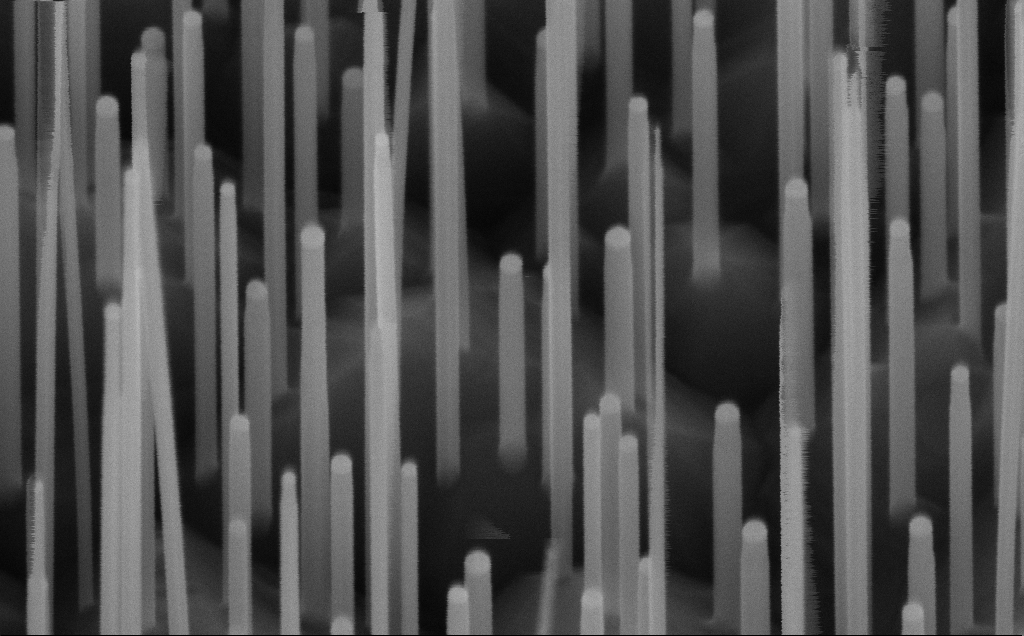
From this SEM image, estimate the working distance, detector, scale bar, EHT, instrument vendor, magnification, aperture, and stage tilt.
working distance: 7 mm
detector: InLens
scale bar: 200 nm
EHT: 10 kV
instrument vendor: Zeiss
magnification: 200 K X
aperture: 30 µm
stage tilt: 45°